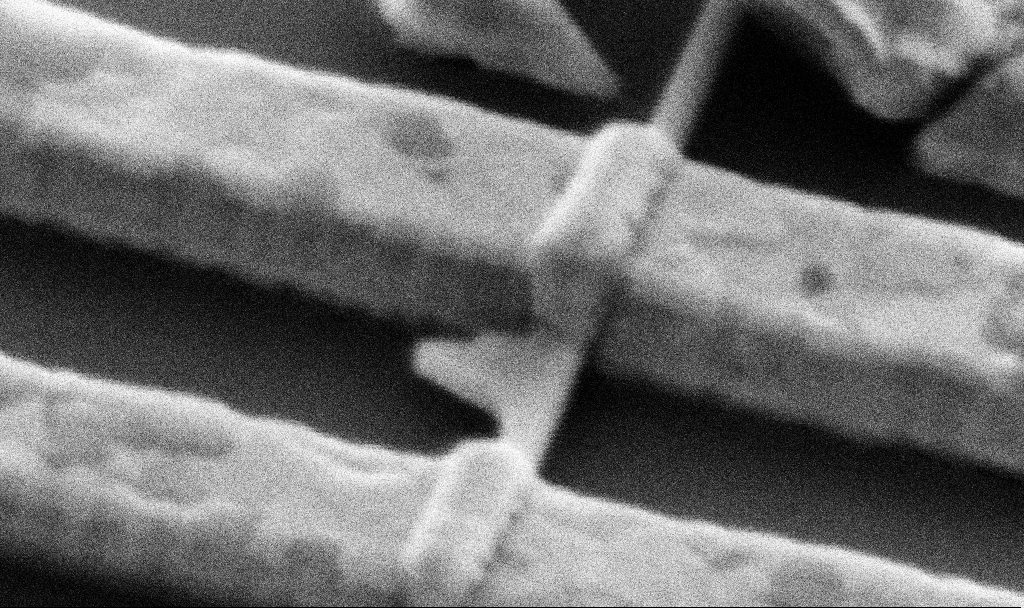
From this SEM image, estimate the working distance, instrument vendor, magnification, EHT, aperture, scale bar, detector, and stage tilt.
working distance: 14.7 mm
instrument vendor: Zeiss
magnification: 150 K X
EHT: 5 kV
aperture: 30 µm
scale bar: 200 nm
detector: SE2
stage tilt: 45°